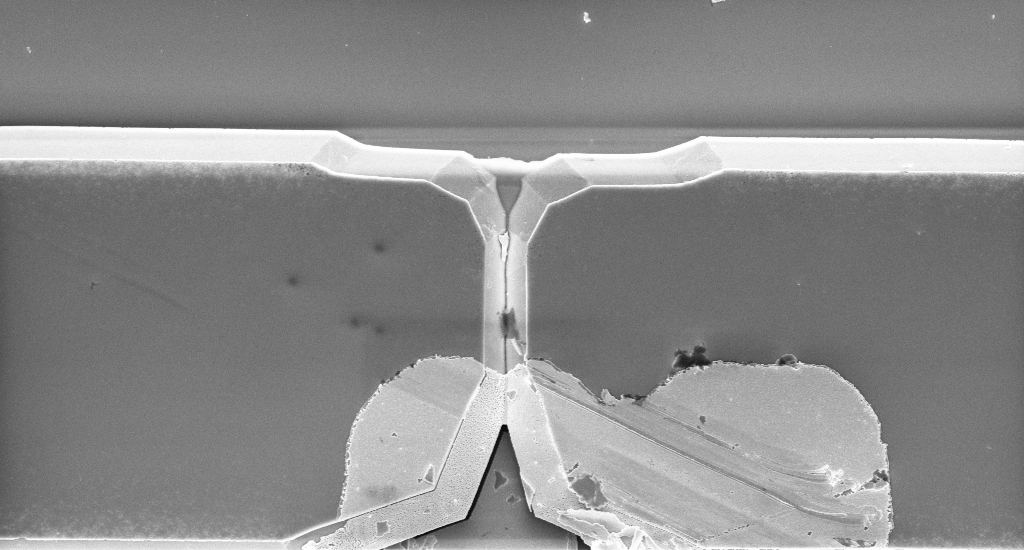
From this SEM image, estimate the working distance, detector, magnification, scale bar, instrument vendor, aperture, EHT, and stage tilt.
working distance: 15 mm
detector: InLens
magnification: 7.5 K X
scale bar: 2000 nm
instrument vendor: Zeiss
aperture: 30 µm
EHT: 5 kV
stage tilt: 0.2°